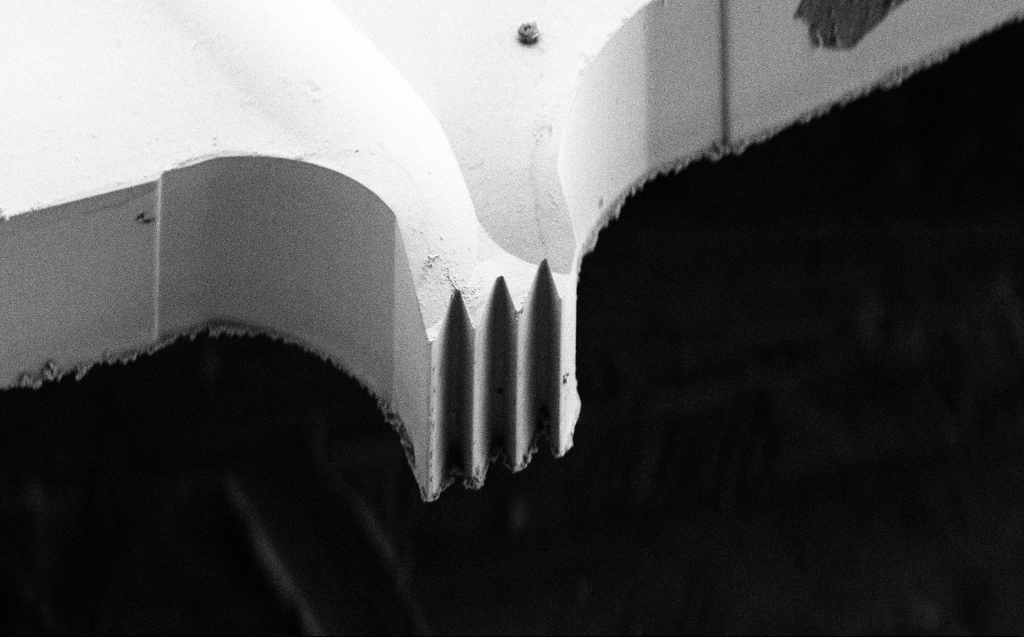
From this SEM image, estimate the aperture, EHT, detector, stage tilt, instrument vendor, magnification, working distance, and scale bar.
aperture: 30 µm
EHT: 5 kV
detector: SE2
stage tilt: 45°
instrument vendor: Zeiss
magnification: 1.51 K X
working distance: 6 mm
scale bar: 20000 nm